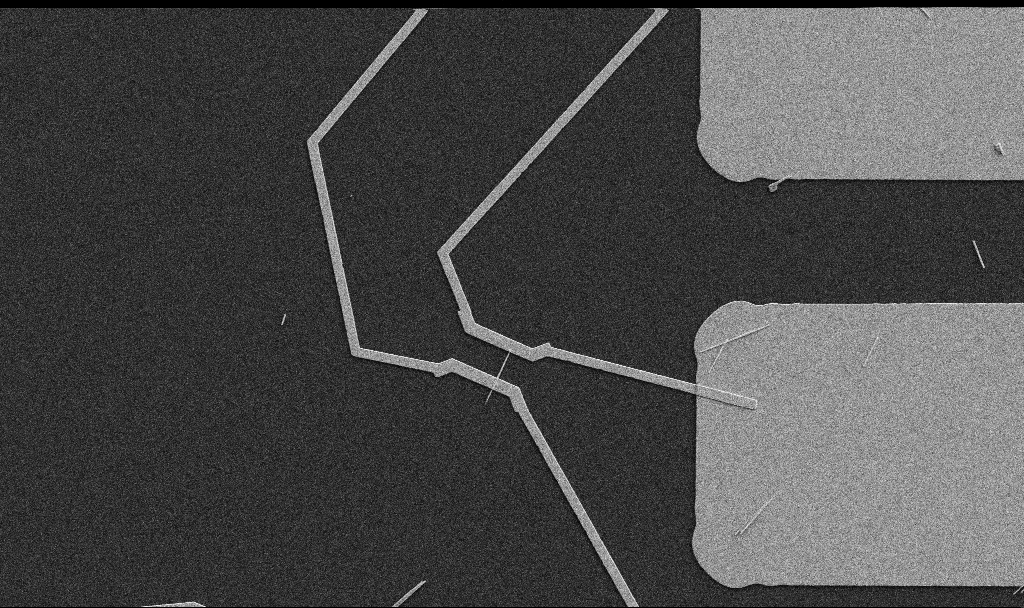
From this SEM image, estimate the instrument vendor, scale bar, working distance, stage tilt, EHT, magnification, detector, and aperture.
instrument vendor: Zeiss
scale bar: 10000 nm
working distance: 10.7 mm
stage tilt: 0°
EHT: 5 kV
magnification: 5 K X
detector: SE2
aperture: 30 µm